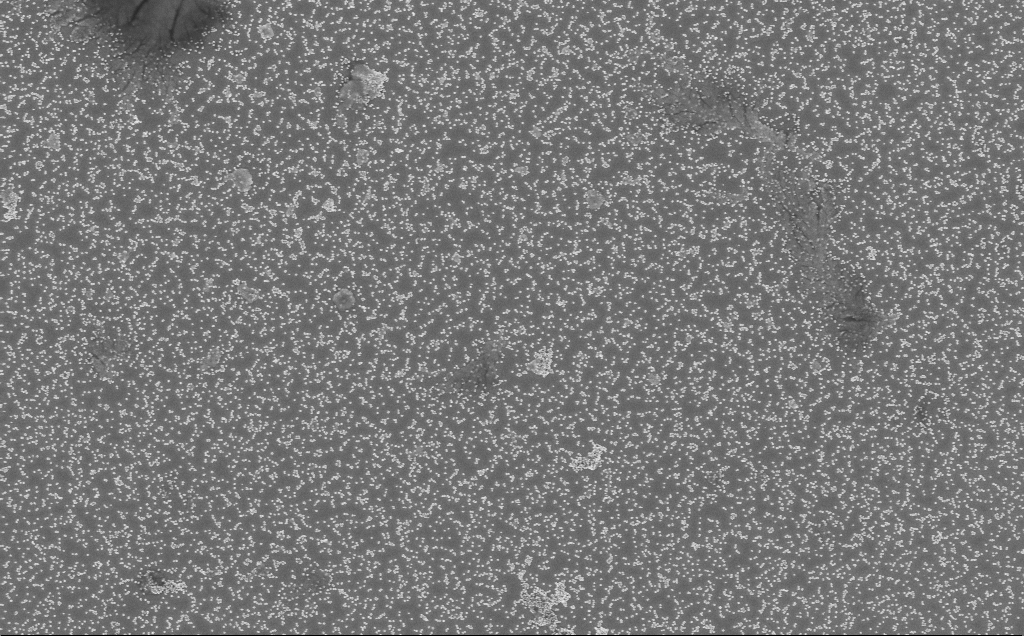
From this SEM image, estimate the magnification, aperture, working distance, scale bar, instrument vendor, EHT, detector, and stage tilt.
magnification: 20 K X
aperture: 30 µm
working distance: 5 mm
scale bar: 1000 nm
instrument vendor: Zeiss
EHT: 3 kV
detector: InLens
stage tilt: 0°